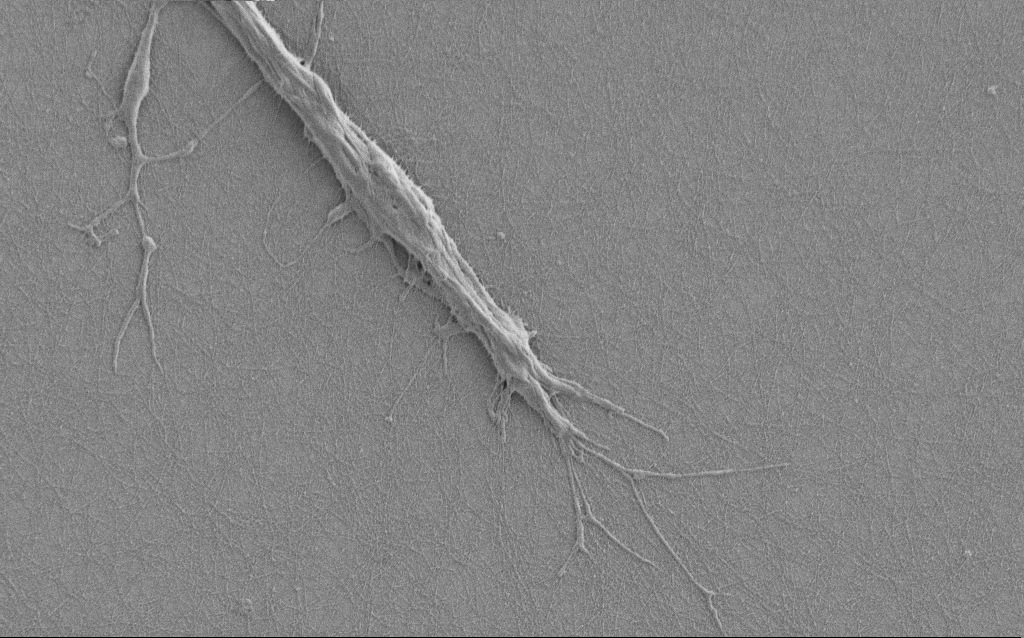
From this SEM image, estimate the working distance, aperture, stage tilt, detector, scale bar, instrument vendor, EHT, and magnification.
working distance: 6 mm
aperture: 30 µm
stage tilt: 0°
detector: SE2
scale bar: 2000 nm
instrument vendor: Zeiss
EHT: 1 kV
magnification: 7.5 K X